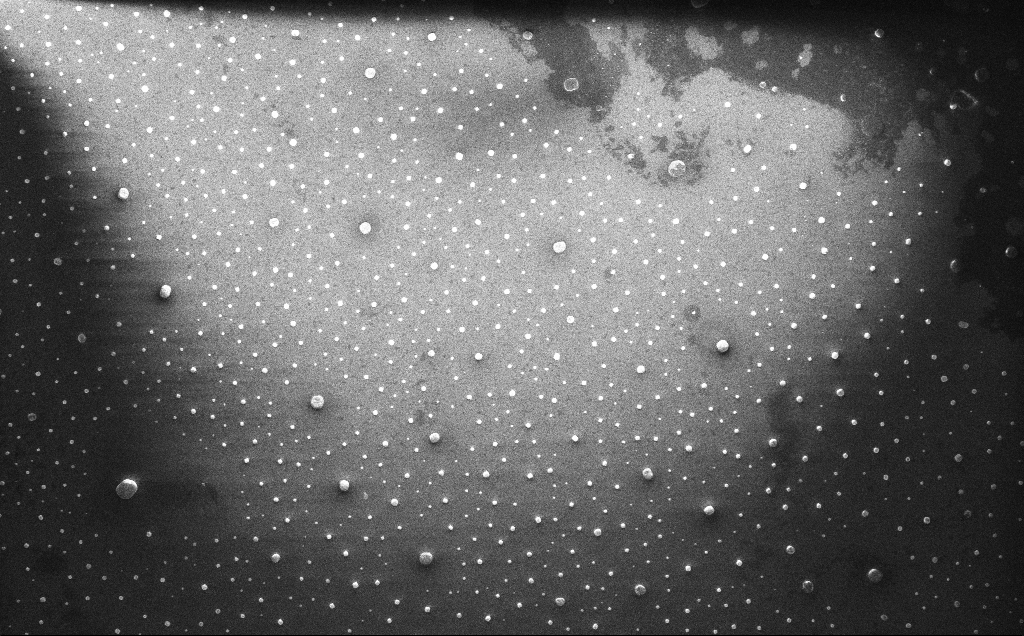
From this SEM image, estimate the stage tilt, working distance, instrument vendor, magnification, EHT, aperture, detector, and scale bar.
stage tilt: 0°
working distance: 5 mm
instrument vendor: Zeiss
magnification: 8.83 K X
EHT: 10 kV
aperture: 30 µm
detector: InLens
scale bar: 2000 nm